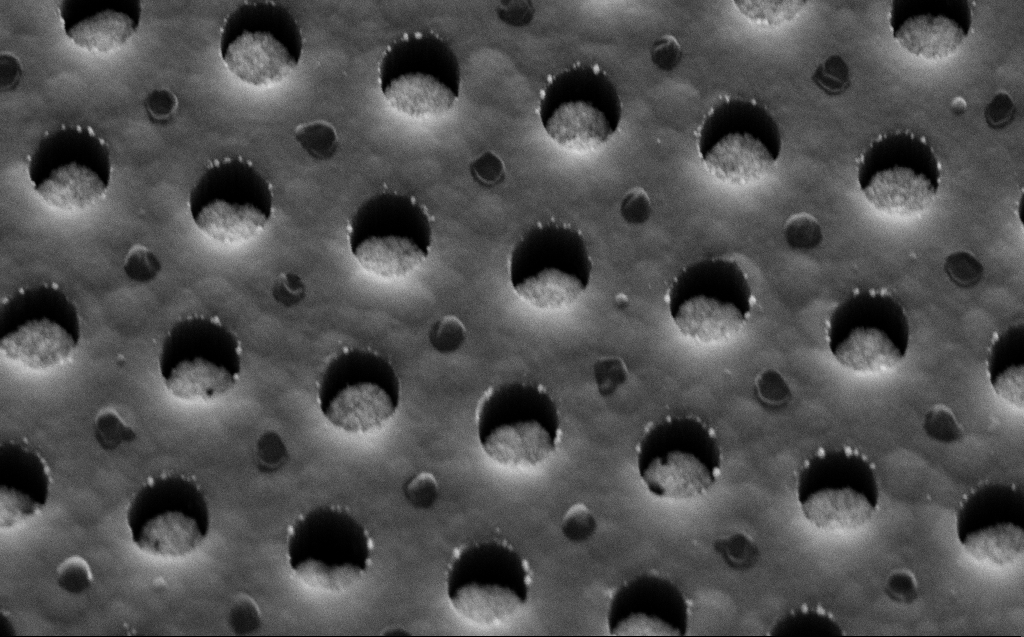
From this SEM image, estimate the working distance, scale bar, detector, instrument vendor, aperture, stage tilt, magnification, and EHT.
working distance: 9 mm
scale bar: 200 nm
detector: SE2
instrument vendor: Zeiss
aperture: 30 µm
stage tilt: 45°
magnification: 74.75 K X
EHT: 5 kV